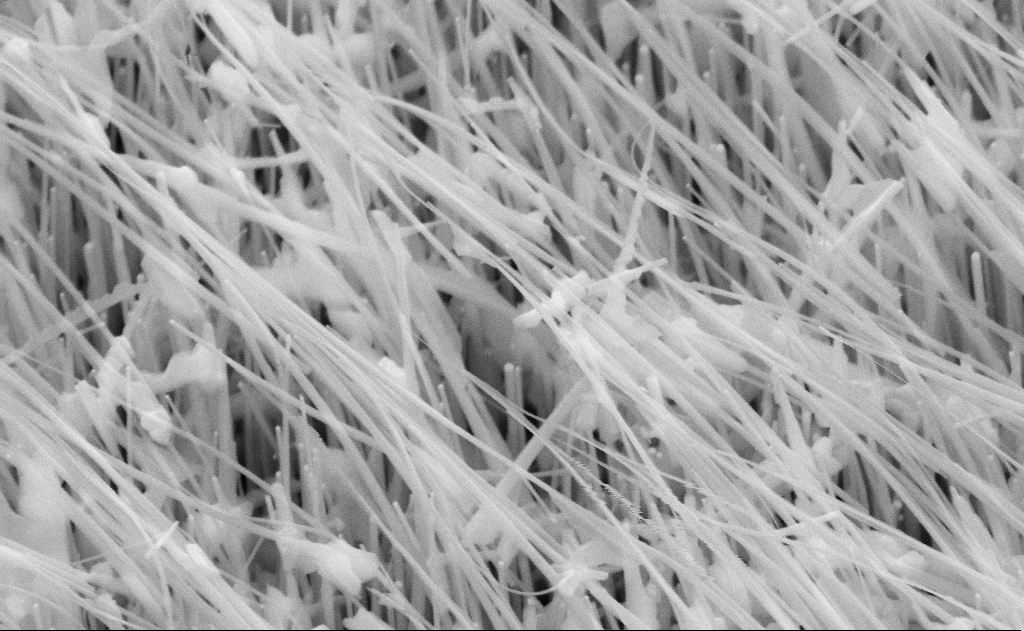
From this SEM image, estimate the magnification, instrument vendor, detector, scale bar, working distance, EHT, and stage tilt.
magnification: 80 K X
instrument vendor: Zeiss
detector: SE2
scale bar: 200 nm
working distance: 12 mm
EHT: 10 kV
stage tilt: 45°